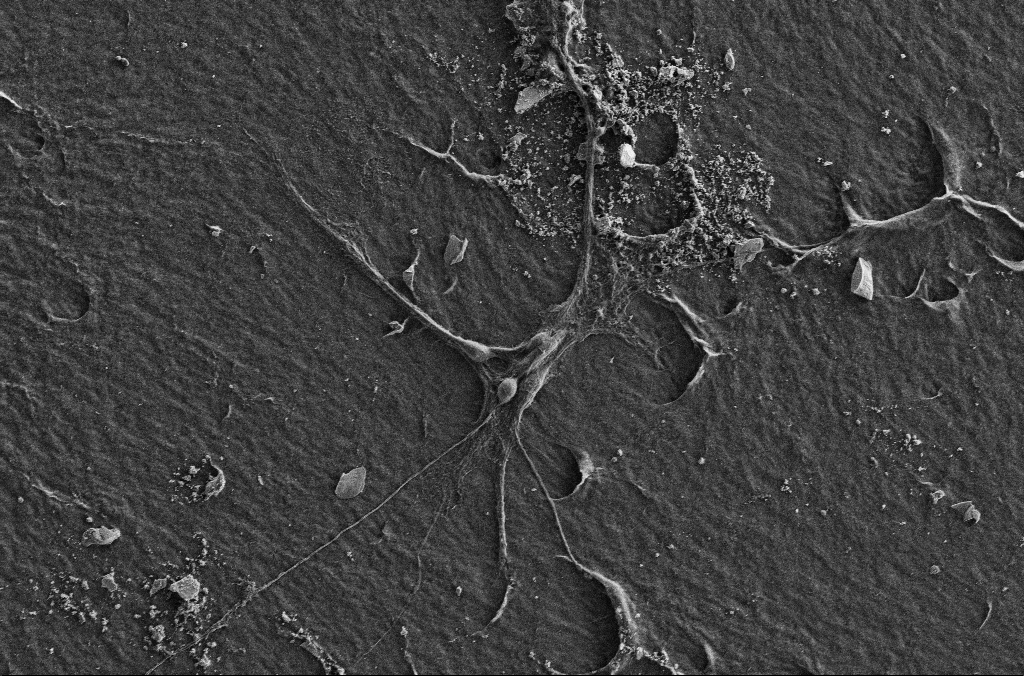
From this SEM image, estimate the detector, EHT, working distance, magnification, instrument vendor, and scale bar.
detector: SE2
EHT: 5 kV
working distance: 2.9 mm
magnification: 1 K X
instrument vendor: Zeiss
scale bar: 20000 nm